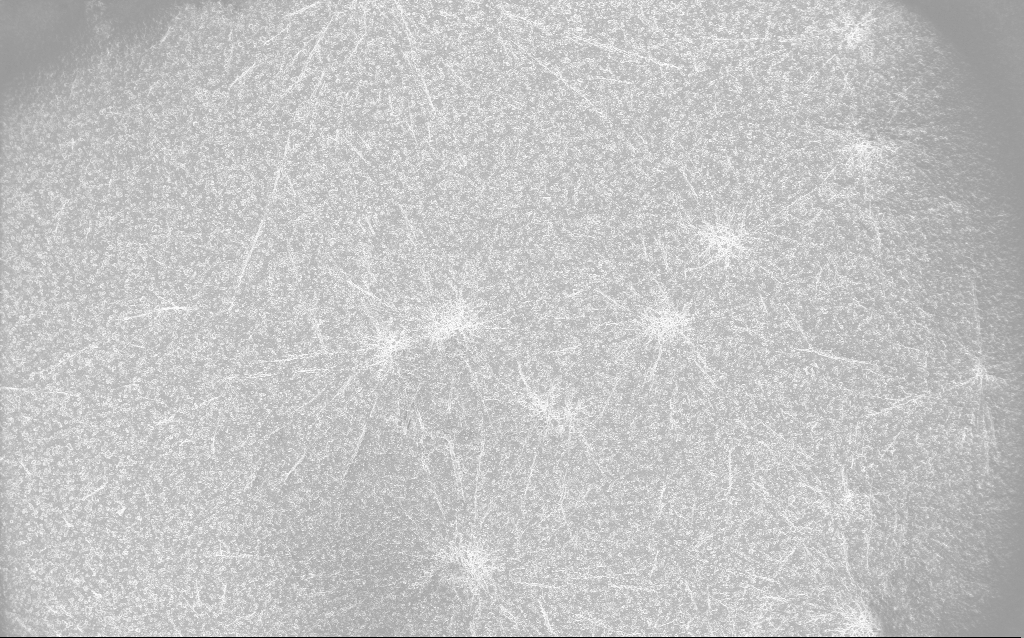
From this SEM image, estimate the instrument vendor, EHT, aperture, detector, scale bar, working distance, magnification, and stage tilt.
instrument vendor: Zeiss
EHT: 10 kV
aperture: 30 µm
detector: InLens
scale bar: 100000 nm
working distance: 2.7 mm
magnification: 0.122 K X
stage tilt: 0°